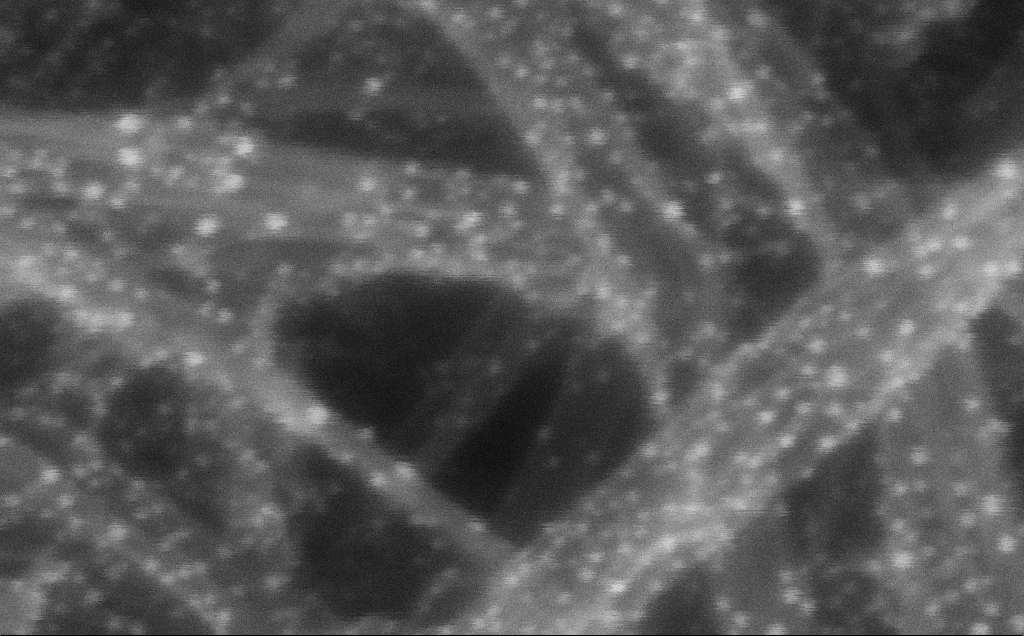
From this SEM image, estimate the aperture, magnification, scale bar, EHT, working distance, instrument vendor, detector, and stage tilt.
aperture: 30 µm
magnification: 1031.08 K X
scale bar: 20 nm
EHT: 10 kV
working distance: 3 mm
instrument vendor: Zeiss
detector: InLens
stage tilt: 0°